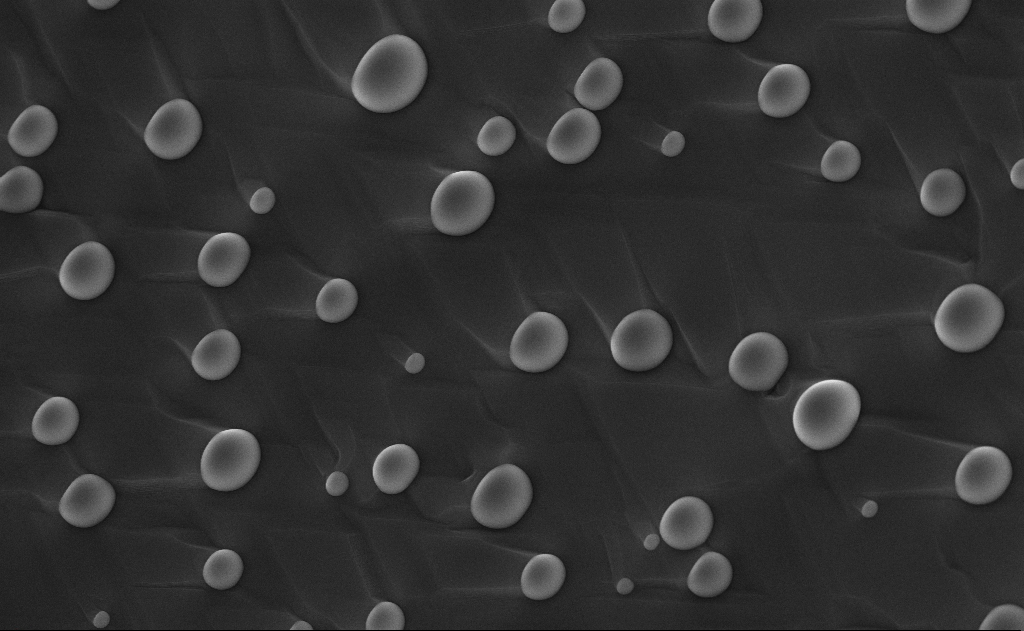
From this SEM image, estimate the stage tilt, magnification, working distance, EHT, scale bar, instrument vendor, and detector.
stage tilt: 0°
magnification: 20 K X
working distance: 11 mm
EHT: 10 kV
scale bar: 2000 nm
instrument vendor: Zeiss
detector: InLens